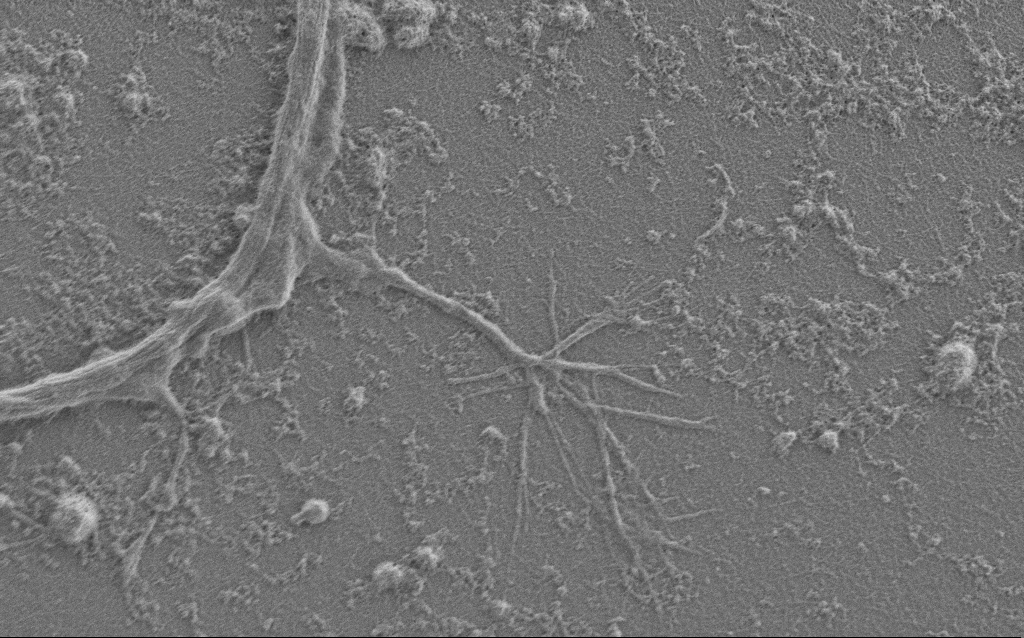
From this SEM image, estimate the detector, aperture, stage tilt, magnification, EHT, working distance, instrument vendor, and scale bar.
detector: SE2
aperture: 30 µm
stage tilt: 0°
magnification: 7.5 K X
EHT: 1 kV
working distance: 6 mm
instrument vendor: Zeiss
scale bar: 2000 nm